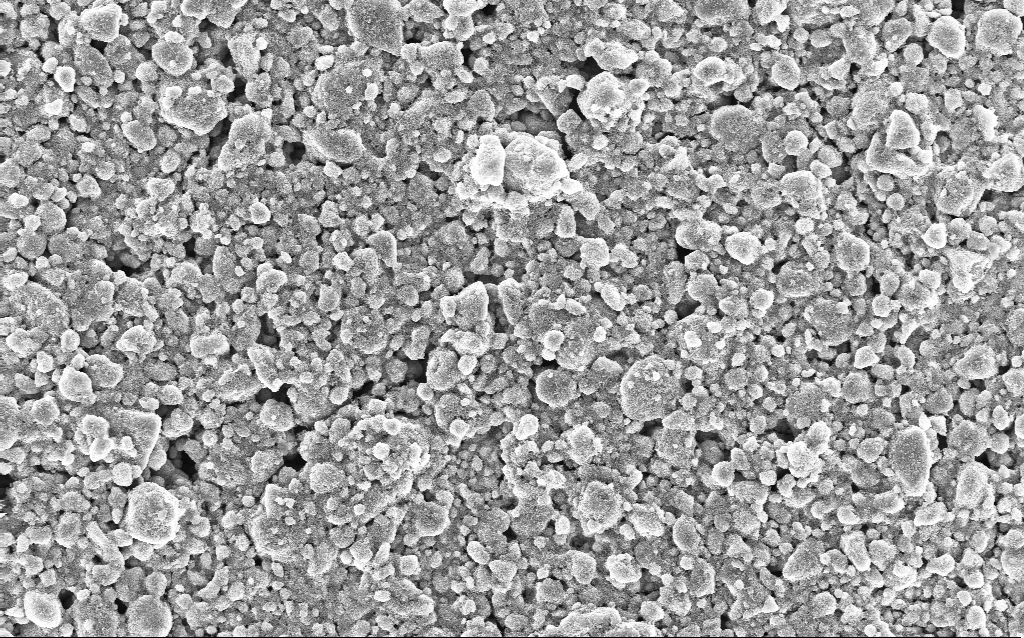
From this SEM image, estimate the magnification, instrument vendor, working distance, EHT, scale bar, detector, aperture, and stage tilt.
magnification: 3.03 K X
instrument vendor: Zeiss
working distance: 3.8 mm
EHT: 10 kV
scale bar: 10000 nm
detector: InLens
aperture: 30 µm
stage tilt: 0°